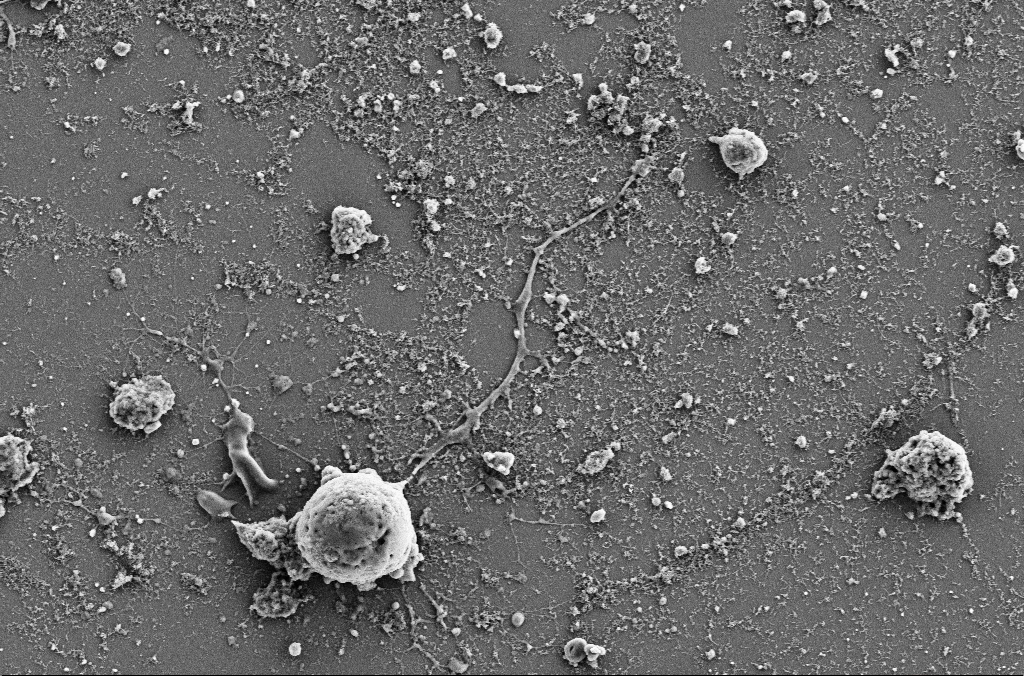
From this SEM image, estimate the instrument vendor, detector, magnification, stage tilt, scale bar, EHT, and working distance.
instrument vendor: Zeiss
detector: SE2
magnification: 4 K X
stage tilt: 0°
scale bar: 10000 nm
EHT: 5 kV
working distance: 4 mm